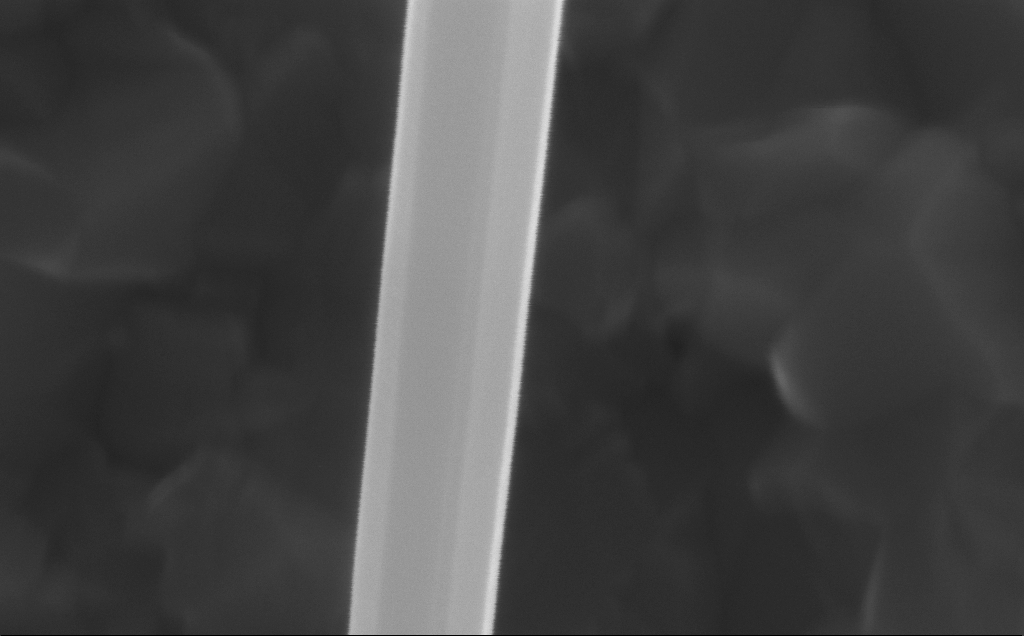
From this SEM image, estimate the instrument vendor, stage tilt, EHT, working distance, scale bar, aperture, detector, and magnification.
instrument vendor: Zeiss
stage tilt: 0°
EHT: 10 kV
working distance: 5 mm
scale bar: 100 nm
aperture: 30 µm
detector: InLens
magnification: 311.25 K X